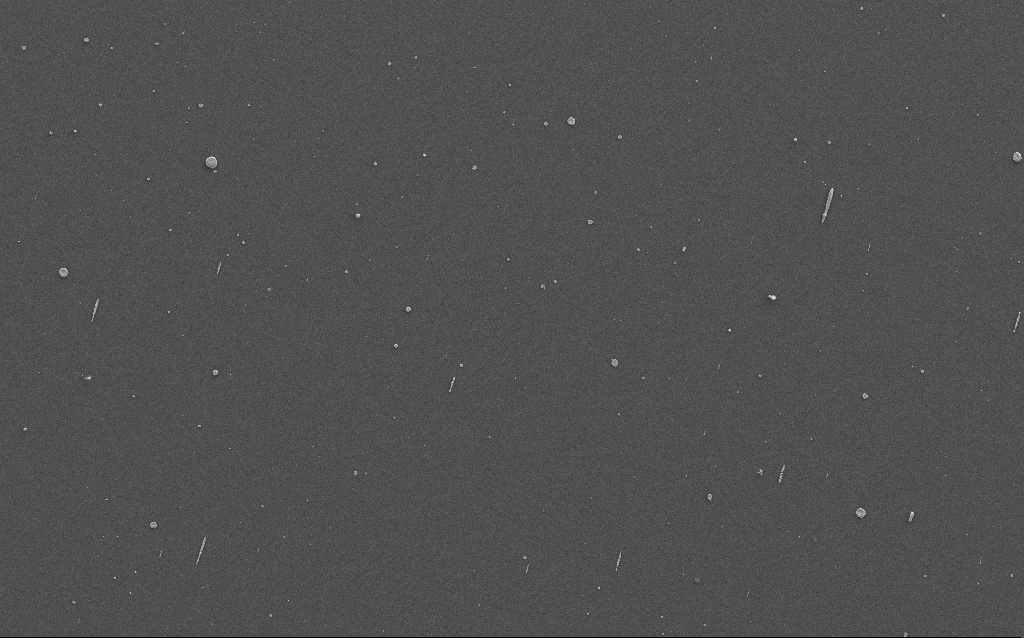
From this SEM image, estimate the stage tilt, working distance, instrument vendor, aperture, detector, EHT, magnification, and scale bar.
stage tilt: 0°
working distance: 4 mm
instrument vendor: Zeiss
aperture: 30 µm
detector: SE2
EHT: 10 kV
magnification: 1.23 K X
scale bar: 20000 nm